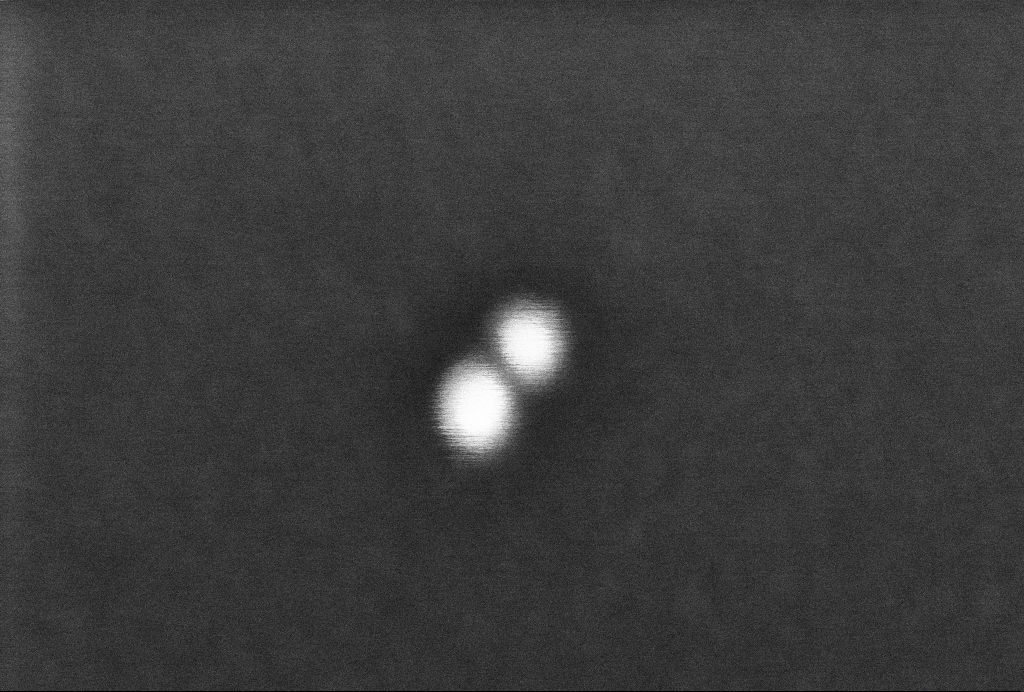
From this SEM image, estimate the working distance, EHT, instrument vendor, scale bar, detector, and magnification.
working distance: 3.3 mm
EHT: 2 kV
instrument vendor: Zeiss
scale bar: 20 nm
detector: InLens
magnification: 935.34 K X